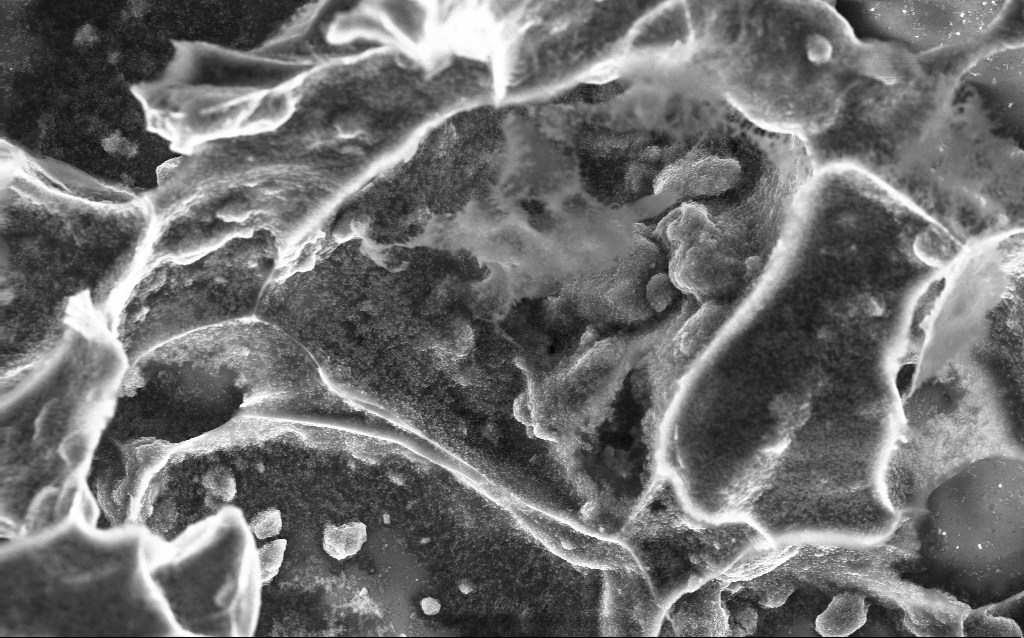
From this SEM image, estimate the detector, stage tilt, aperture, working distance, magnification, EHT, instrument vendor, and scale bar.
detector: InLens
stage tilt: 0°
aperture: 30 µm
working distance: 2.8 mm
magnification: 5.83 K X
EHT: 10 kV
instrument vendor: Zeiss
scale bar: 10000 nm